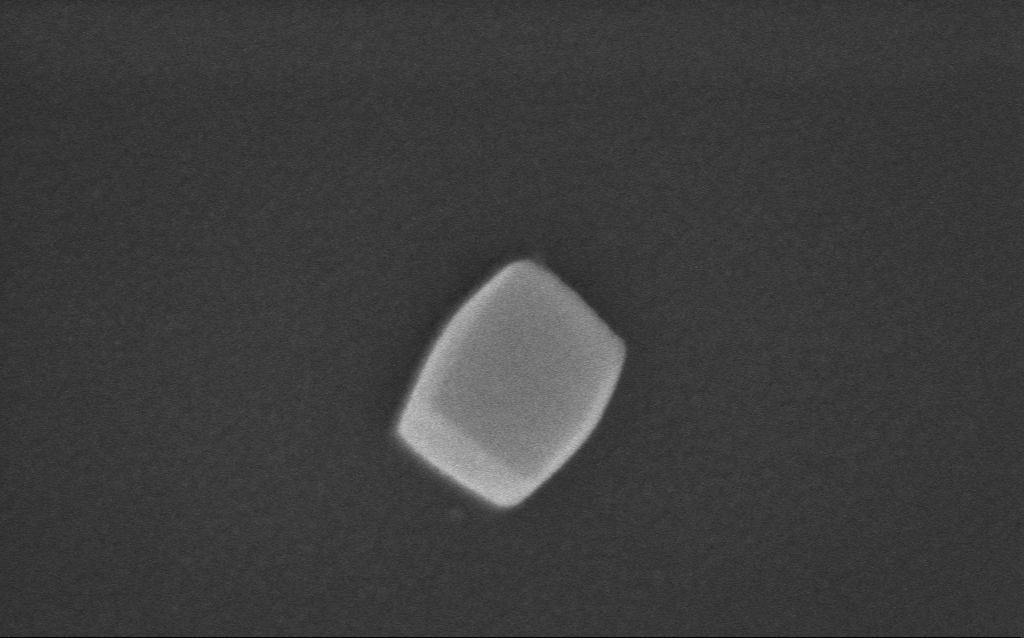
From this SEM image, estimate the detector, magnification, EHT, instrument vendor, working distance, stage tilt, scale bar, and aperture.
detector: InLens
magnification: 222.51 K X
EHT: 10 kV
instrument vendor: Zeiss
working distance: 5 mm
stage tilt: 0°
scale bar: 200 nm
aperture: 30 µm